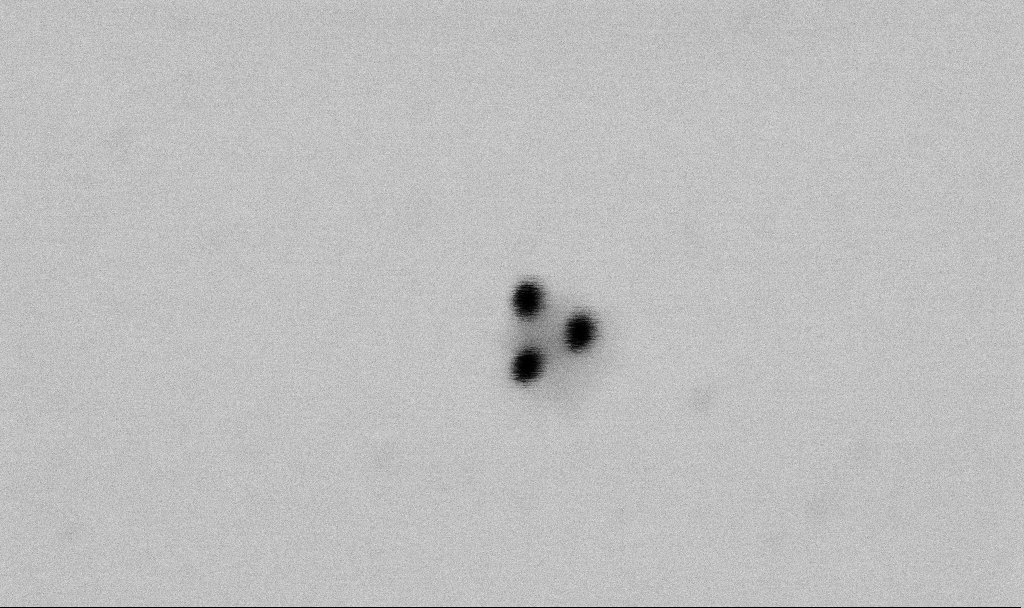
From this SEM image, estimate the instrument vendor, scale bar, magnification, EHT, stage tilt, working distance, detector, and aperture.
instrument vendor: Zeiss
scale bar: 100 nm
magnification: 400 K X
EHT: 2 kV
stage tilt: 0°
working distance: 6.5 mm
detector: SE2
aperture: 30 µm